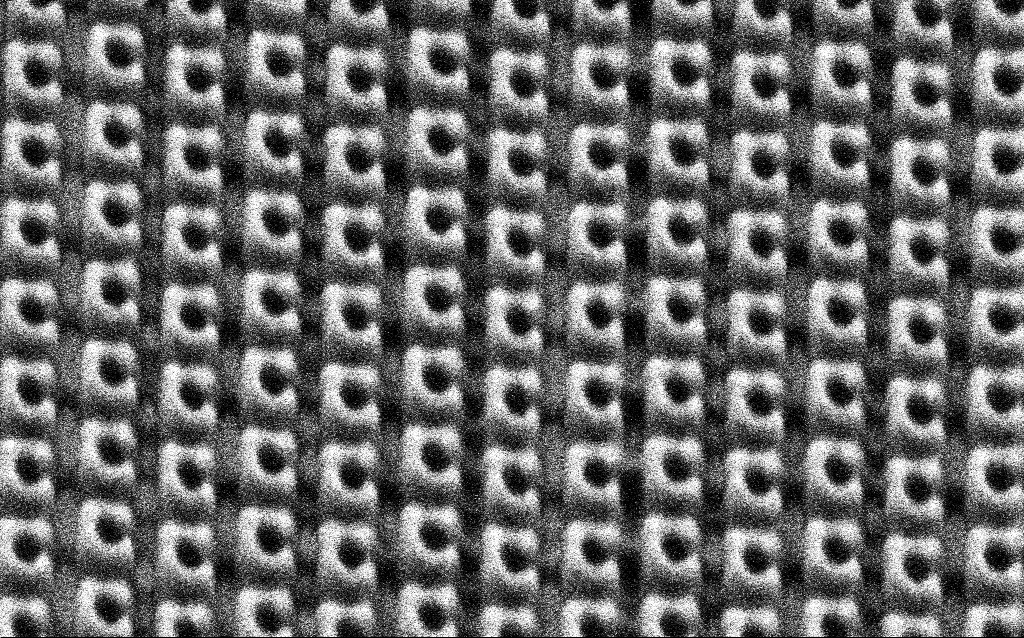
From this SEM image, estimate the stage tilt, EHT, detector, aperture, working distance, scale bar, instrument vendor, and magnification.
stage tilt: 30°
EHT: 1.5 kV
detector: SE2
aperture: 30 µm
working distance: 7.3 mm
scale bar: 1000 nm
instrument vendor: Zeiss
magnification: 63.13 K X